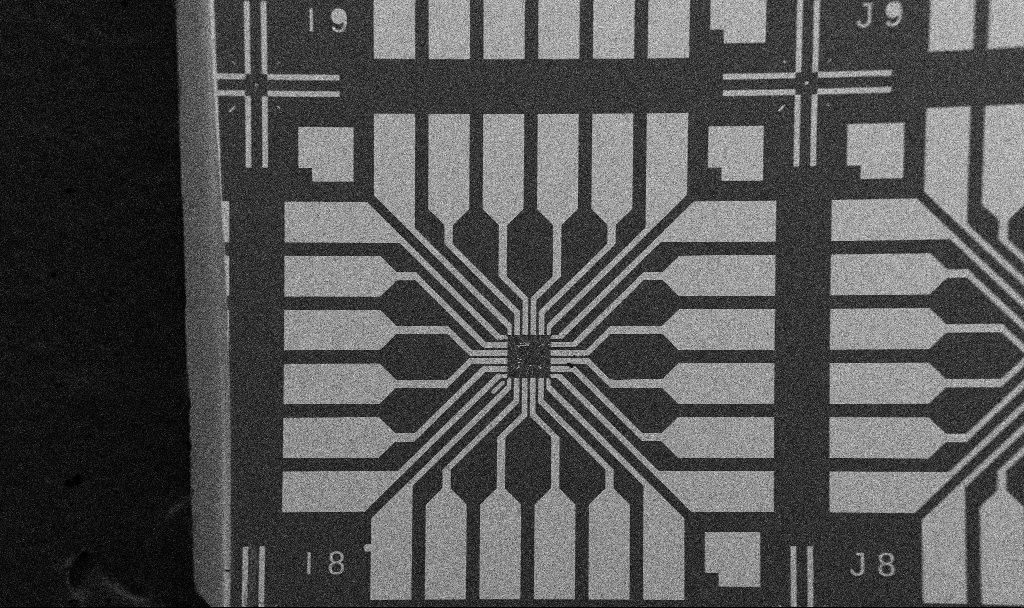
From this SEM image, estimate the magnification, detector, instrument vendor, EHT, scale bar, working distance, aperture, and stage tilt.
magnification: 0.1 K X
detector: SE2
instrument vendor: Zeiss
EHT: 5 kV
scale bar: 200000 nm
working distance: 10.7 mm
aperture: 30 µm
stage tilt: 0°